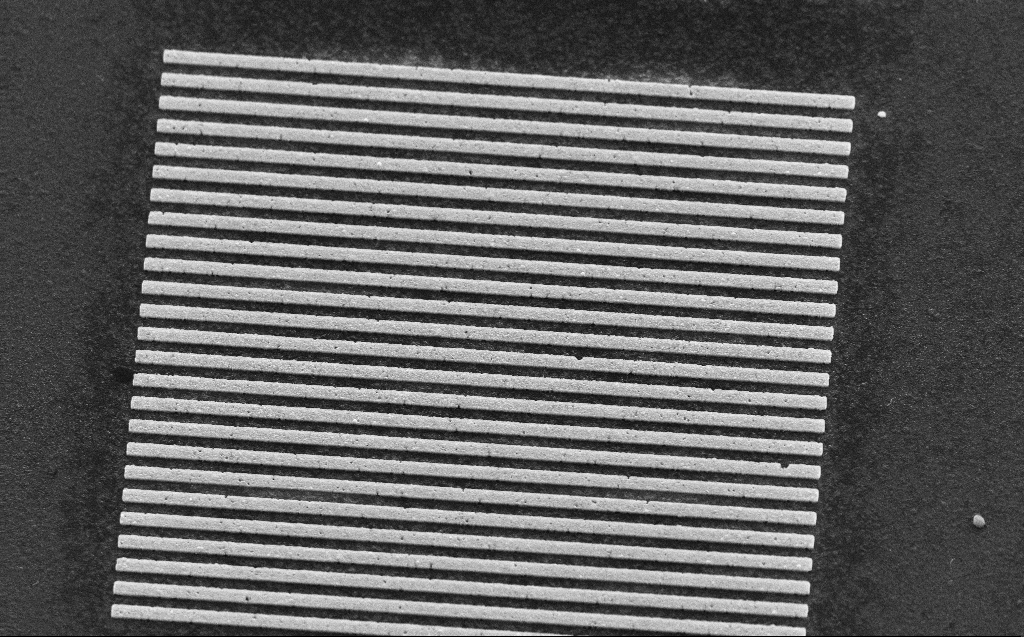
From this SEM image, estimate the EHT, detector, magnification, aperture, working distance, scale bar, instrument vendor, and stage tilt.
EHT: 30 kV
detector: SE2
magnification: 8.69 K X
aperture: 30 µm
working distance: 5 mm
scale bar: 2000 nm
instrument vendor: Zeiss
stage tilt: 45°